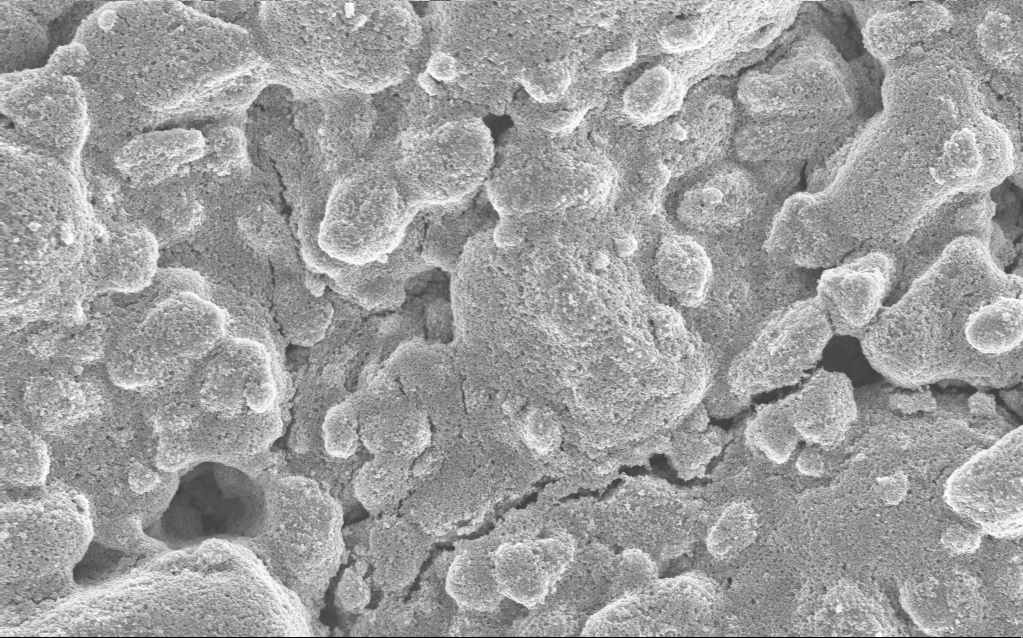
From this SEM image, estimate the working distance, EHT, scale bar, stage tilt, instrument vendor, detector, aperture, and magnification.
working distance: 4.9 mm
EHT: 5 kV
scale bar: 1000 nm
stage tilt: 0°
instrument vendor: Zeiss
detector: InLens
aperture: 30 µm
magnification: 14.07 K X